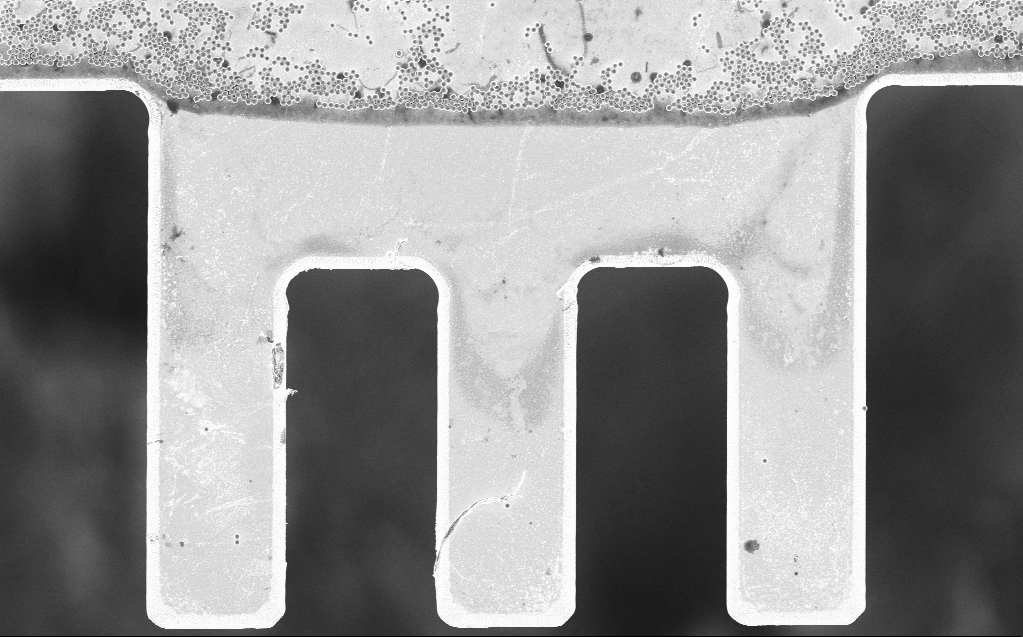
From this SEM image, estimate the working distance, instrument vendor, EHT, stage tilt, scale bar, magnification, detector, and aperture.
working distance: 7 mm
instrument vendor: Zeiss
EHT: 3 kV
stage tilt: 0°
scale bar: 20000 nm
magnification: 2.66 K X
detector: InLens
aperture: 30 µm